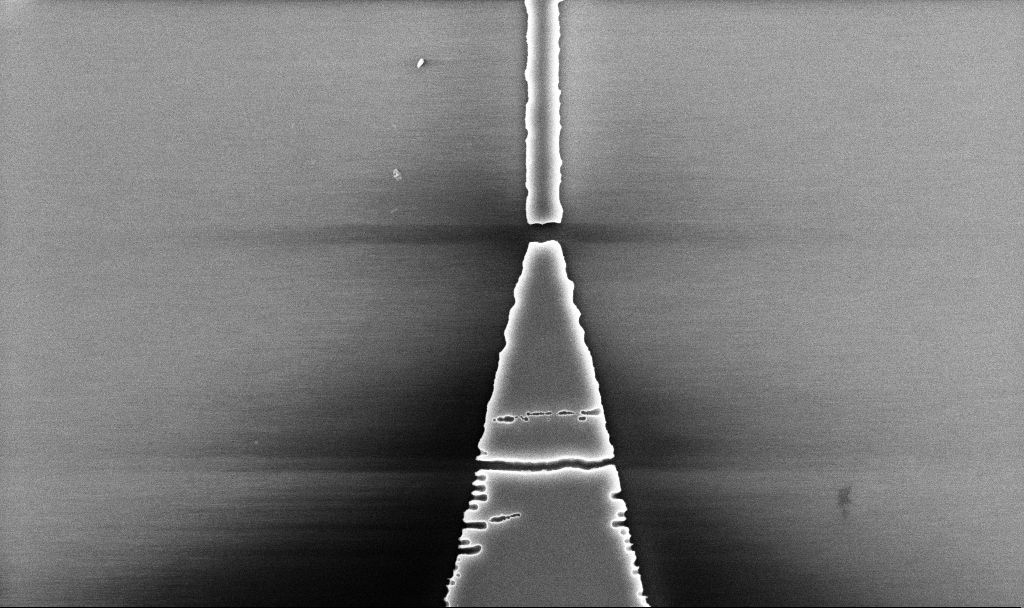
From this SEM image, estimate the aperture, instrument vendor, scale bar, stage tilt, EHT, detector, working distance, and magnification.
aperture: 30 µm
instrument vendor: Zeiss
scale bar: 2000 nm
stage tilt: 0°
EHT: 5 kV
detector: InLens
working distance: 5.2 mm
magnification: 22.93 K X